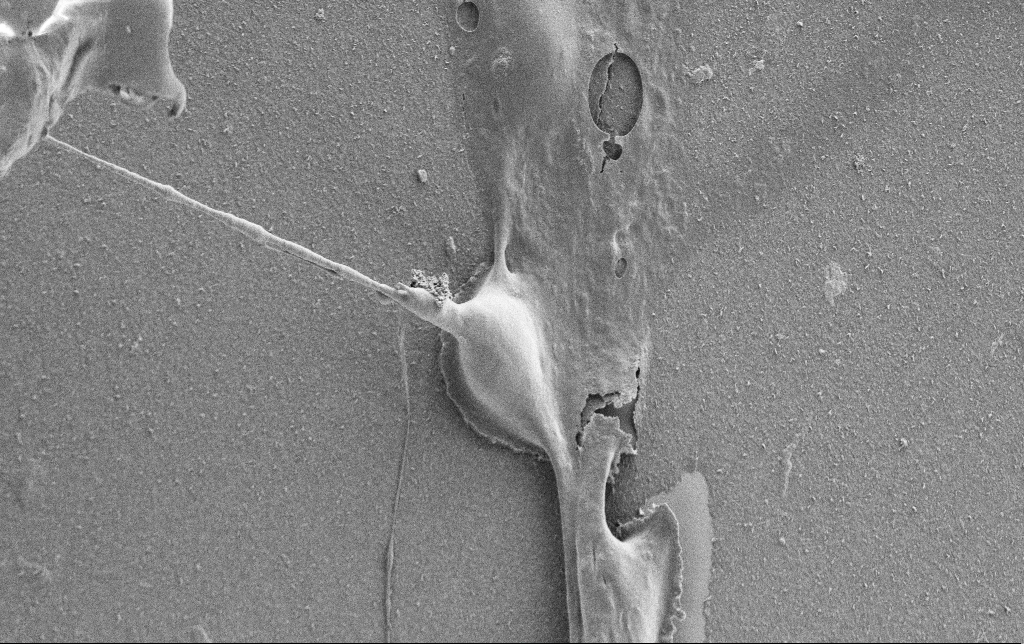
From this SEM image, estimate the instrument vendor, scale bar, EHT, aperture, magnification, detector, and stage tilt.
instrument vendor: Zeiss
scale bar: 10000 nm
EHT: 0.9 kV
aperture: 30 µm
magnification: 5 K X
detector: SE2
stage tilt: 0°